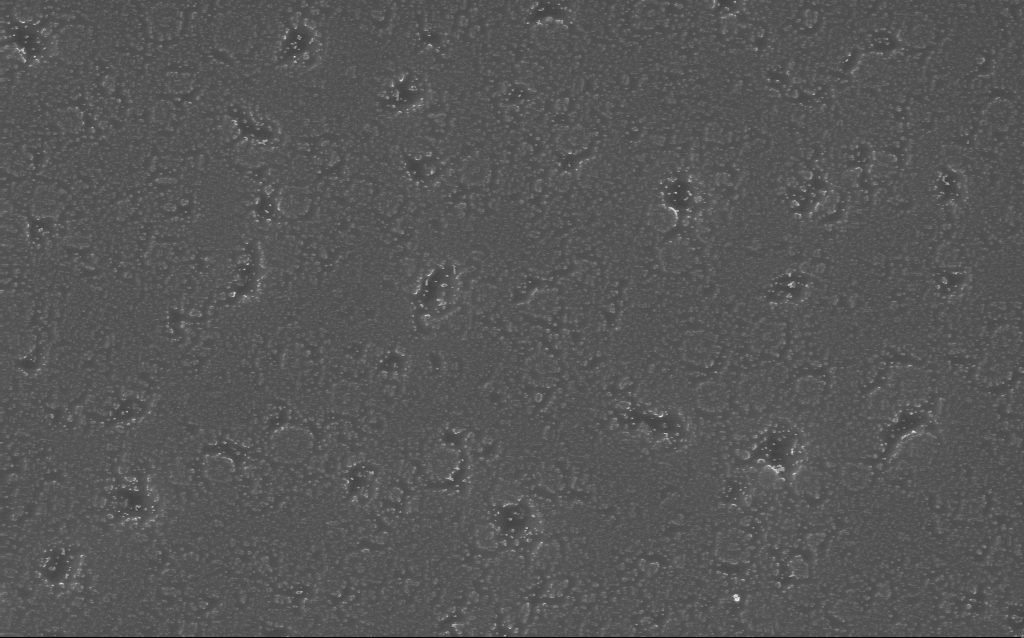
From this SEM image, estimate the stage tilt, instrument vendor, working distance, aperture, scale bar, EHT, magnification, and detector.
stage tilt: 0°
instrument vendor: Zeiss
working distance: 5 mm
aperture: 30 µm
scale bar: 2000 nm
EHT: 10 kV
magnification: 32.35 K X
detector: InLens